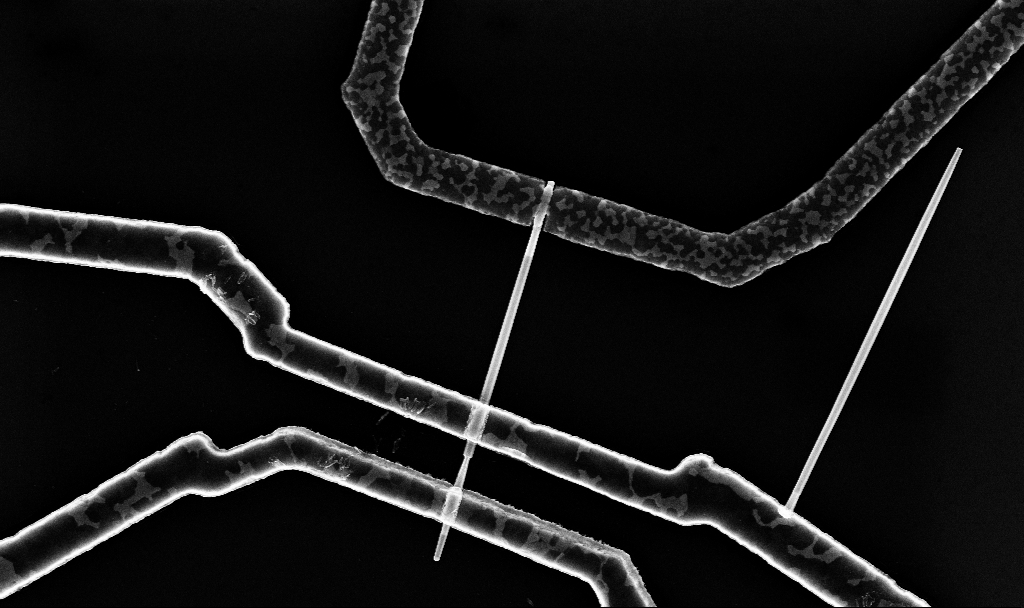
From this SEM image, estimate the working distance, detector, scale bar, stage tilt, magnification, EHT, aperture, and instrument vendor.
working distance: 7.7 mm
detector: InLens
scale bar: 2000 nm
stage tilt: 0°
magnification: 25.28 K X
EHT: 10 kV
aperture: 30 µm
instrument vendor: Zeiss